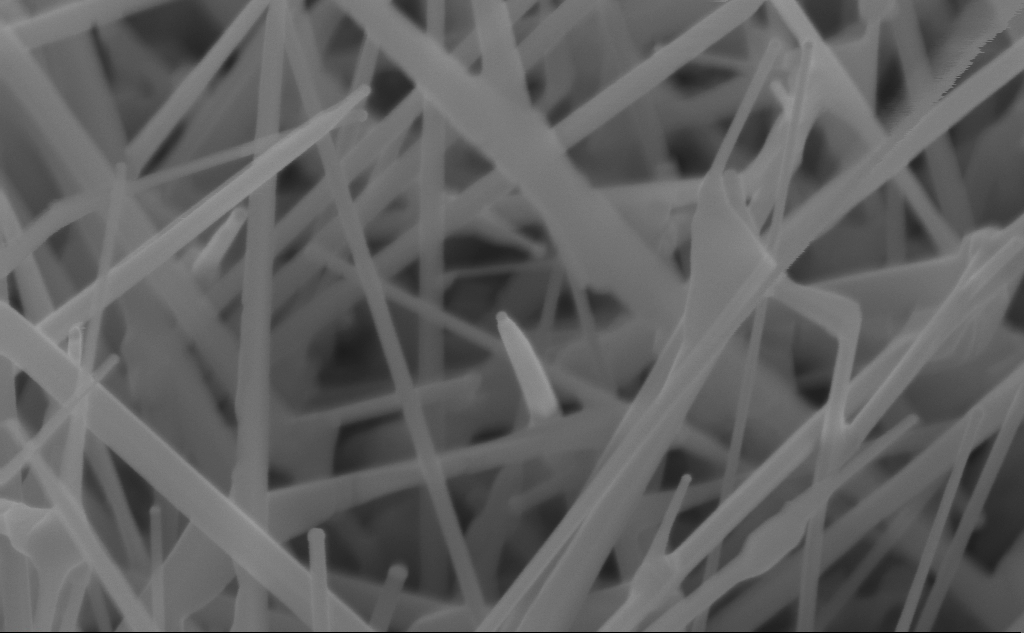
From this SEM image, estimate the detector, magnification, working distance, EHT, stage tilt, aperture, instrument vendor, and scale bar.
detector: InLens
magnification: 150 K X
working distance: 4 mm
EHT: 10 kV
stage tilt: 45°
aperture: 30 µm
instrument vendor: Zeiss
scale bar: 200 nm